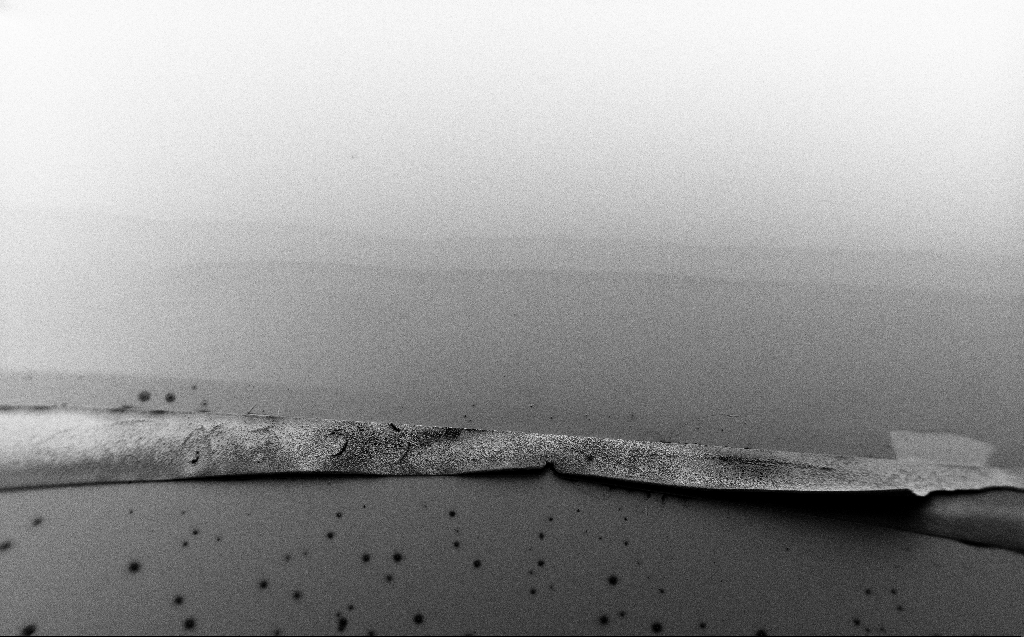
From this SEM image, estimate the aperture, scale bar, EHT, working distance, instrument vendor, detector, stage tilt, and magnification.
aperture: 30 µm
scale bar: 200000 nm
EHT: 5 kV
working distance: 9 mm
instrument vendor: Zeiss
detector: SE2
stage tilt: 45.1°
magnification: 0.072 K X